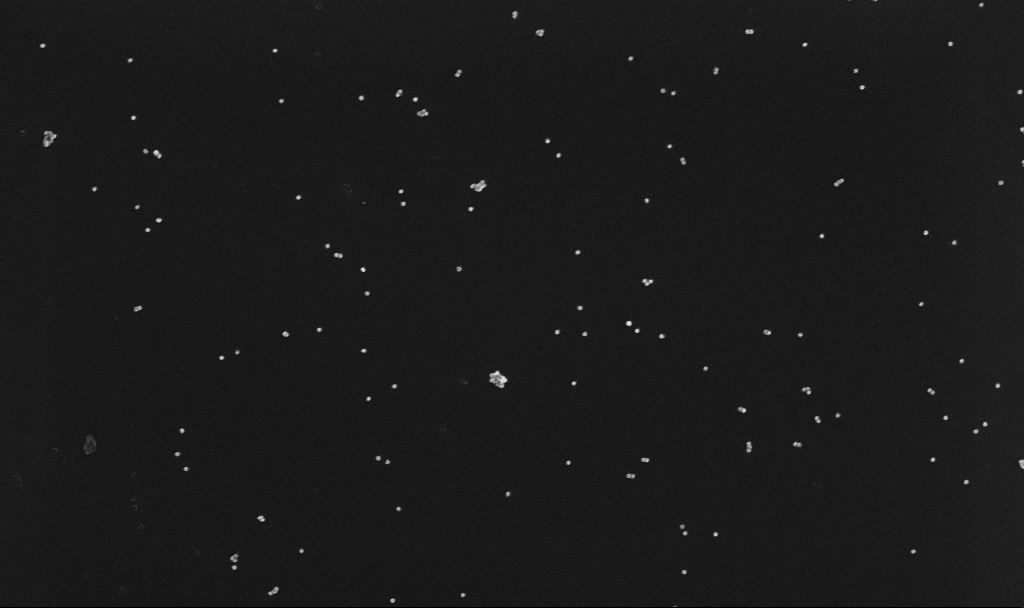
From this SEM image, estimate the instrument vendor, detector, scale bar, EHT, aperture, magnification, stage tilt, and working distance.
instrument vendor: Zeiss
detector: InLens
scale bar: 1000 nm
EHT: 10 kV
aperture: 30 µm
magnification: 70 K X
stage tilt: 0°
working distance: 3.4 mm